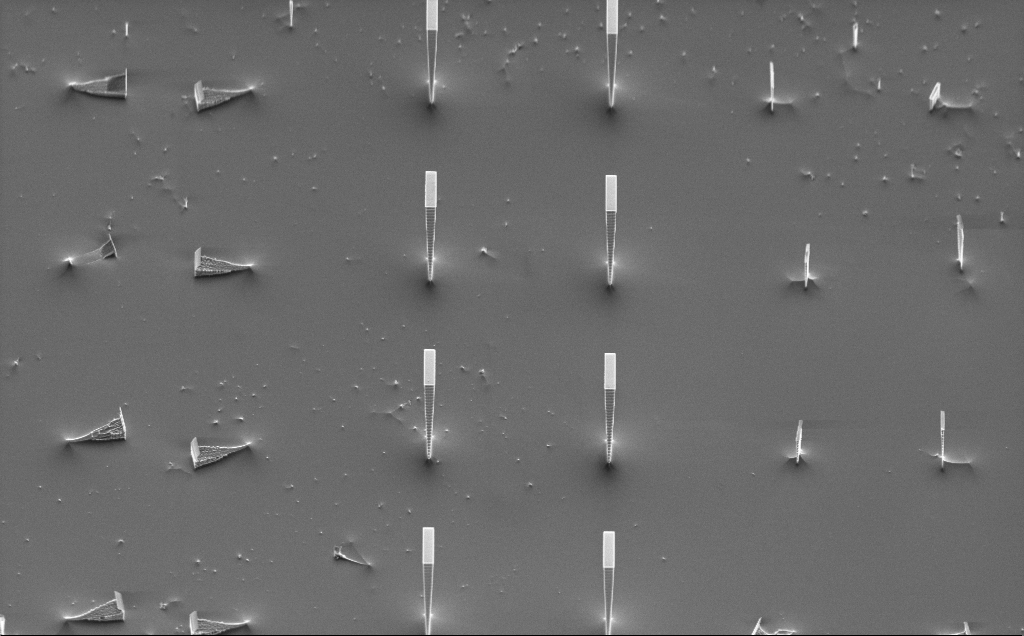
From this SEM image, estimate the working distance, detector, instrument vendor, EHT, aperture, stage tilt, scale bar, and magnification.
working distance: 16 mm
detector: SE2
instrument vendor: Zeiss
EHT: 5 kV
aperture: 30 µm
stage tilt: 50°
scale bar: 10000 nm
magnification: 1.33 K X